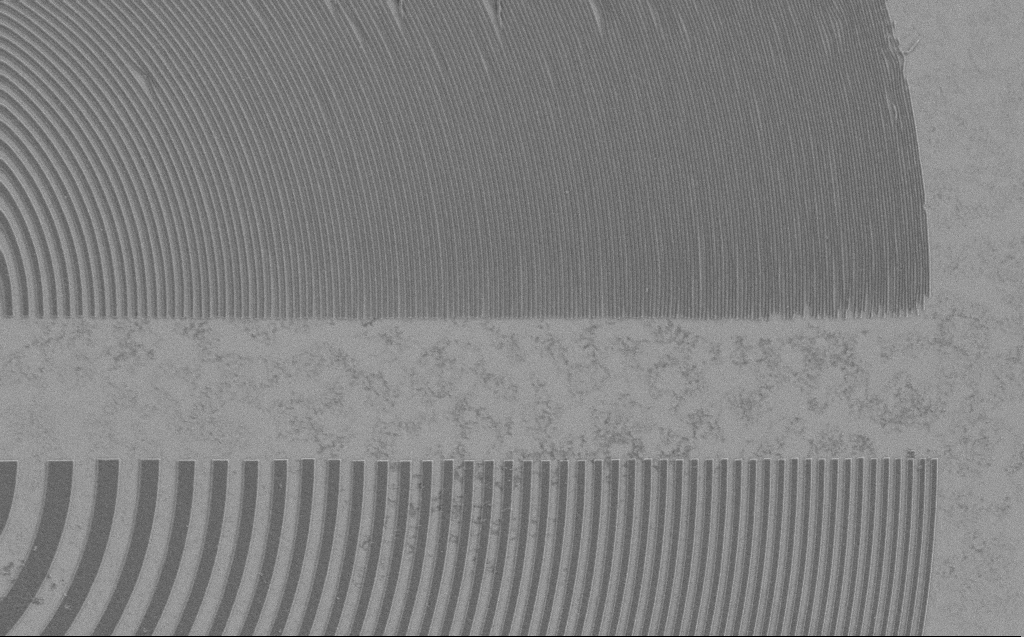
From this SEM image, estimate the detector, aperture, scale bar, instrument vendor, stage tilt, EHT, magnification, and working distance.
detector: SE2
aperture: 30 µm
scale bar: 10000 nm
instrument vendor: Zeiss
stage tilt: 0°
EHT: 1.2 kV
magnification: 5.54 K X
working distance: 5 mm